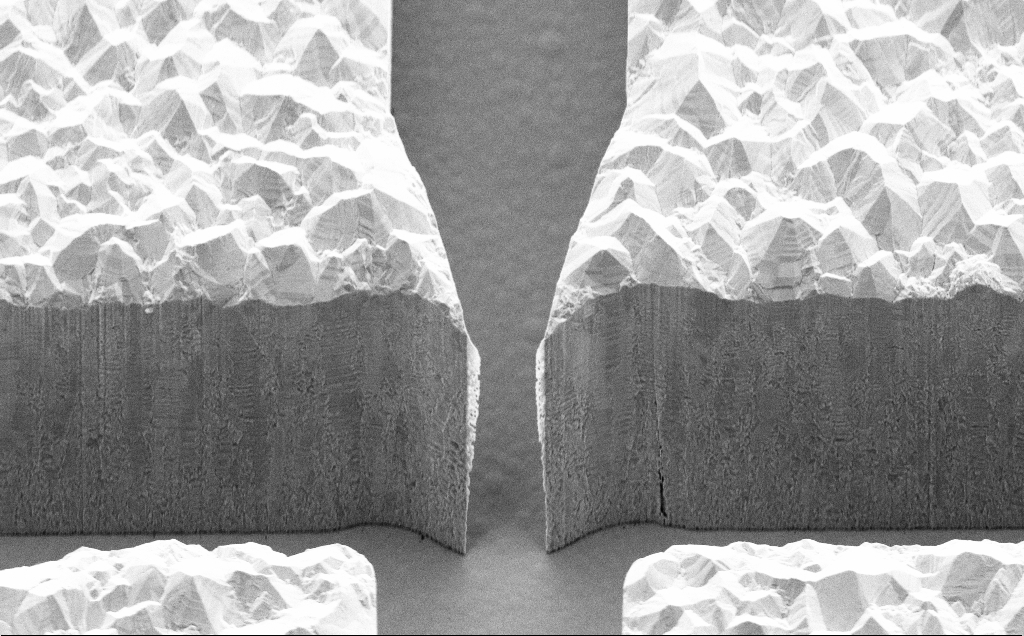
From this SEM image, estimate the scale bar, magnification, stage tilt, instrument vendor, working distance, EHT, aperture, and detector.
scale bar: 2000 nm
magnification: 8.83 K X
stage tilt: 45°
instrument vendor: Zeiss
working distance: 10 mm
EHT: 5 kV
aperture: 30 µm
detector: SE2